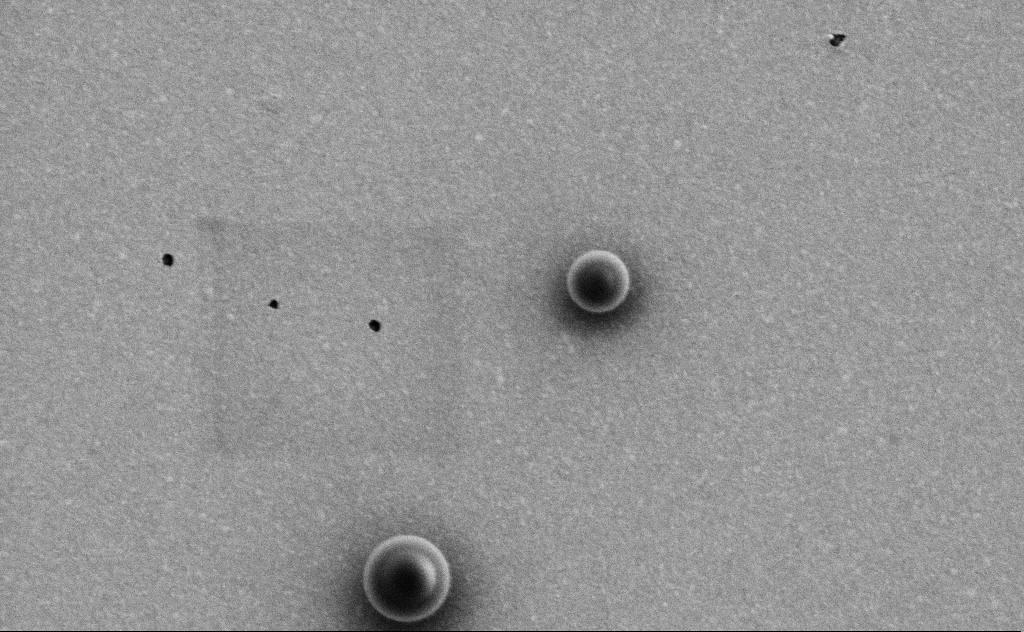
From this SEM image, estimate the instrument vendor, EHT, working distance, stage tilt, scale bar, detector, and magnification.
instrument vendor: Zeiss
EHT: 3 kV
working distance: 10 mm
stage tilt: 0°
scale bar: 1000 nm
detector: SE2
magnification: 43.9 K X